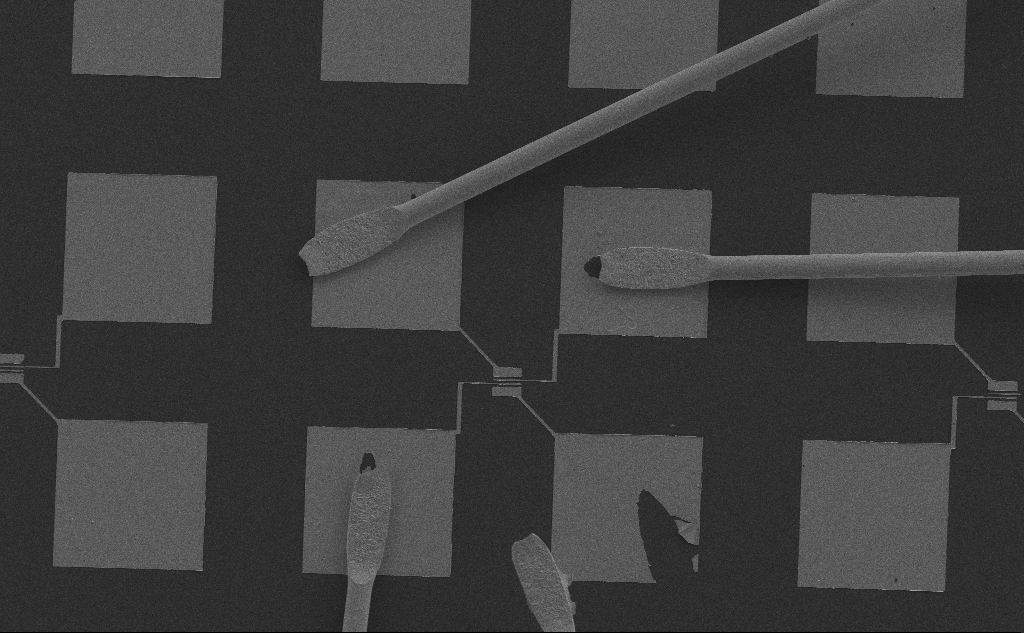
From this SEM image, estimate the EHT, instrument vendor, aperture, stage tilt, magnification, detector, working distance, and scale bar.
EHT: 10 kV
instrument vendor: Zeiss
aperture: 30 µm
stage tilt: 0°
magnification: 0.368 K X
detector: SE2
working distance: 6 mm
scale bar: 100000 nm